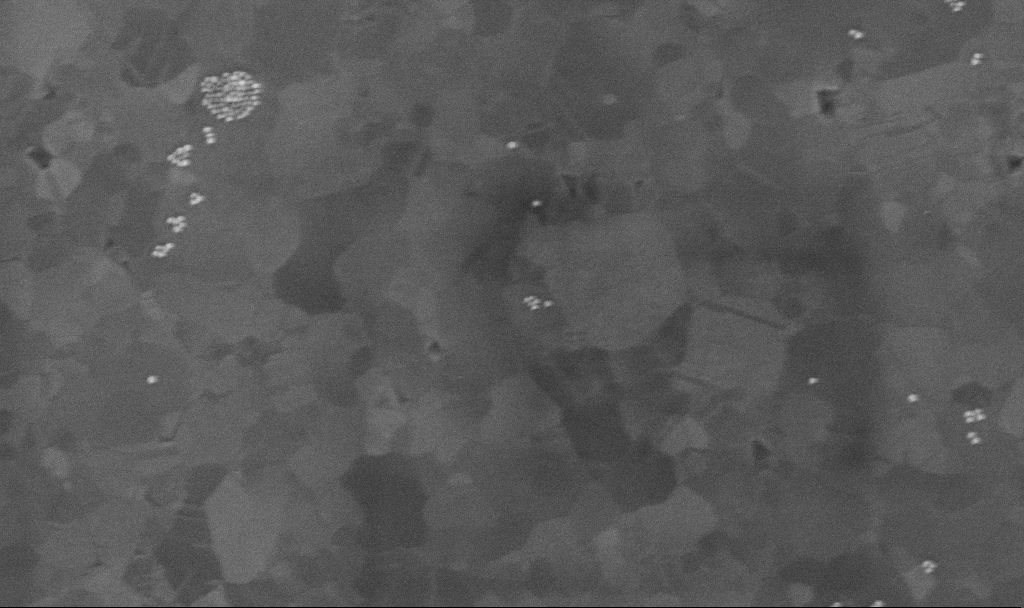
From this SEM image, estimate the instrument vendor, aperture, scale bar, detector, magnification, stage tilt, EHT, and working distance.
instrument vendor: Zeiss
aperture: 30 µm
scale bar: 200 nm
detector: InLens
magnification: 127.01 K X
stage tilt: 0°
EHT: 10 kV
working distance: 3.4 mm